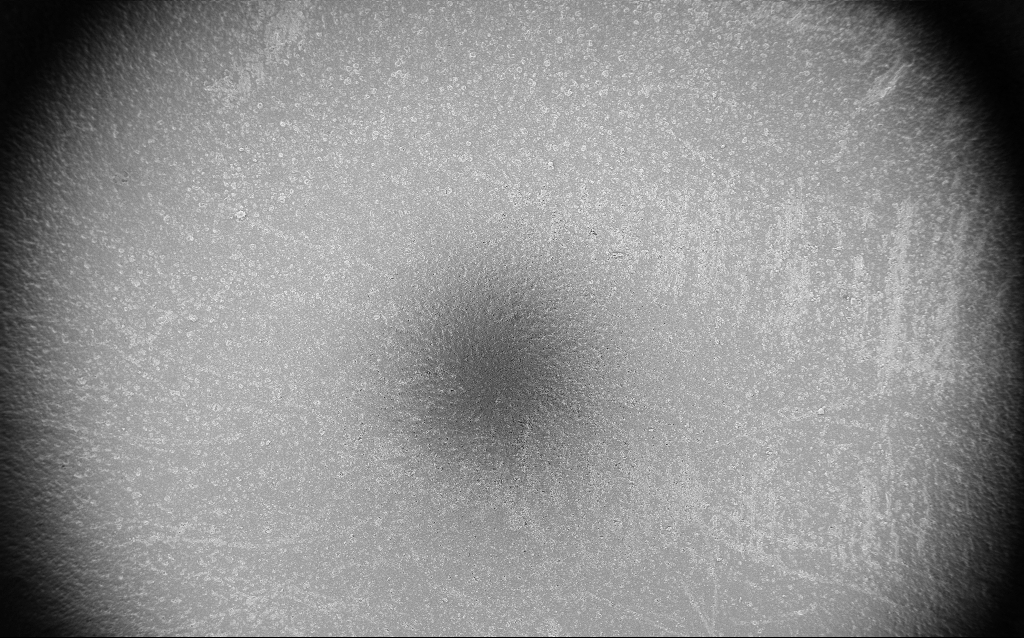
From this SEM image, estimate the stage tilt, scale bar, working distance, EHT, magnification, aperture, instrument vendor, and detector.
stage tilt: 0°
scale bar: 200000 nm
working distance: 2.9 mm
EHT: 5 kV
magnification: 0.1 K X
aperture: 30 µm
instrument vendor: Zeiss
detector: InLens